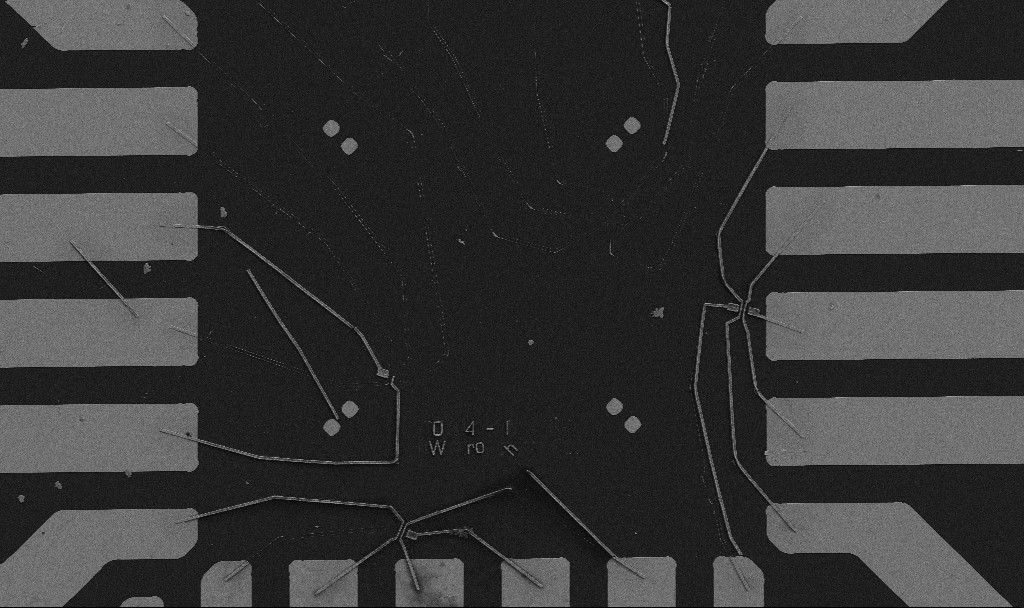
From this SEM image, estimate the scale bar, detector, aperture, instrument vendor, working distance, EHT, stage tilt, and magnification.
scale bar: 20000 nm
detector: SE2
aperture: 30 µm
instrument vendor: Zeiss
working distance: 9.7 mm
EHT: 5 kV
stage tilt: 0°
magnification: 1.3 K X